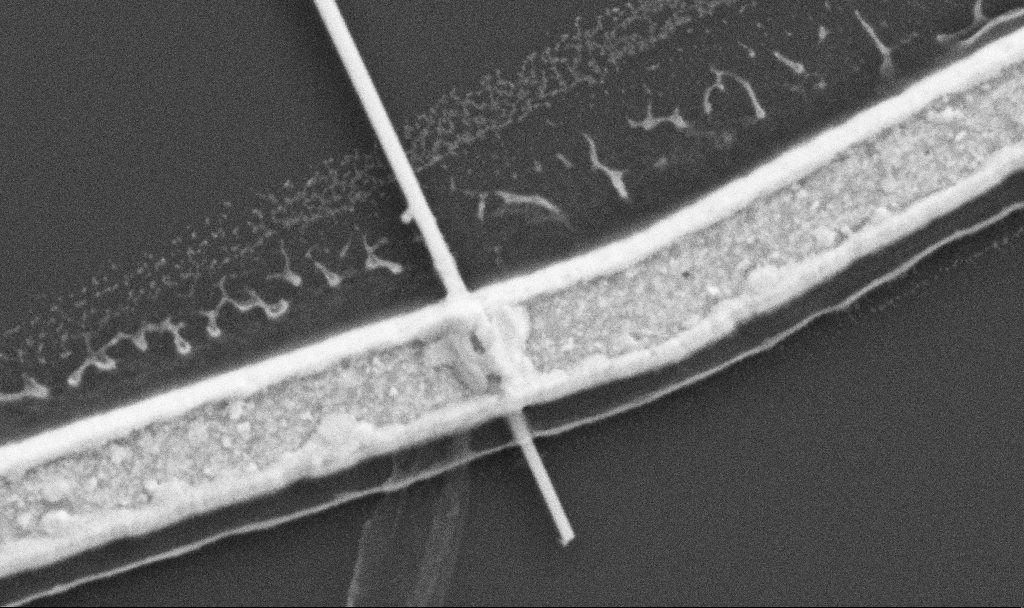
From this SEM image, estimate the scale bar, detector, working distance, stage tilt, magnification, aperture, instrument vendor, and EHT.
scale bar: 1000 nm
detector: SE2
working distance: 10.7 mm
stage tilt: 0°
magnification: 65.97 K X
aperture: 30 µm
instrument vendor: Zeiss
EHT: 5 kV